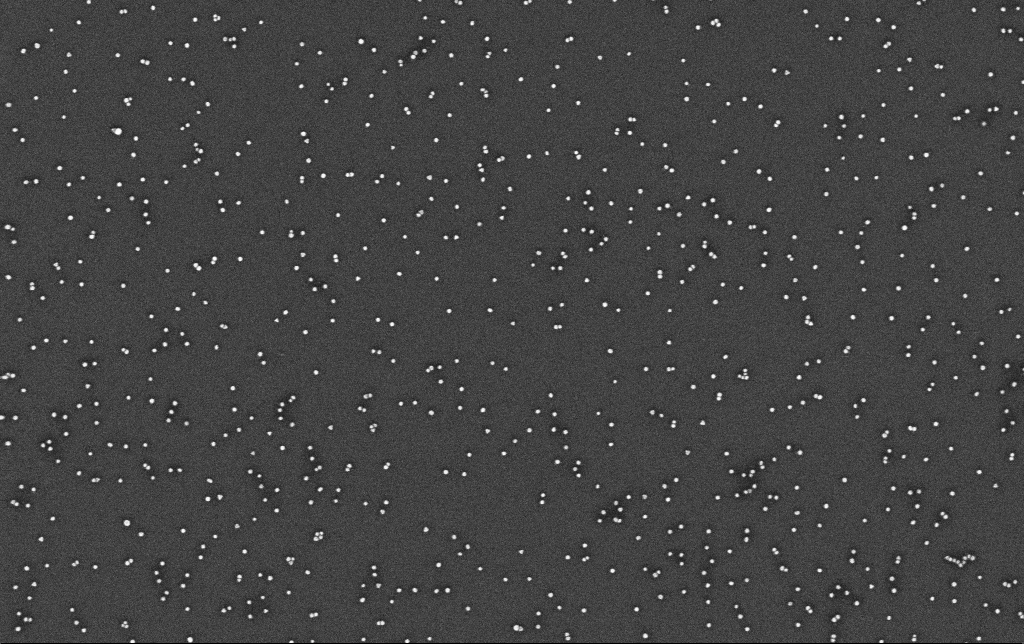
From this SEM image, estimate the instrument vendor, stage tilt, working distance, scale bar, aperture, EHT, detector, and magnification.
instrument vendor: Zeiss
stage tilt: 0°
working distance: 3.3 mm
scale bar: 200 nm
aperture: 30 µm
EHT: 10 kV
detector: InLens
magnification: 100 K X